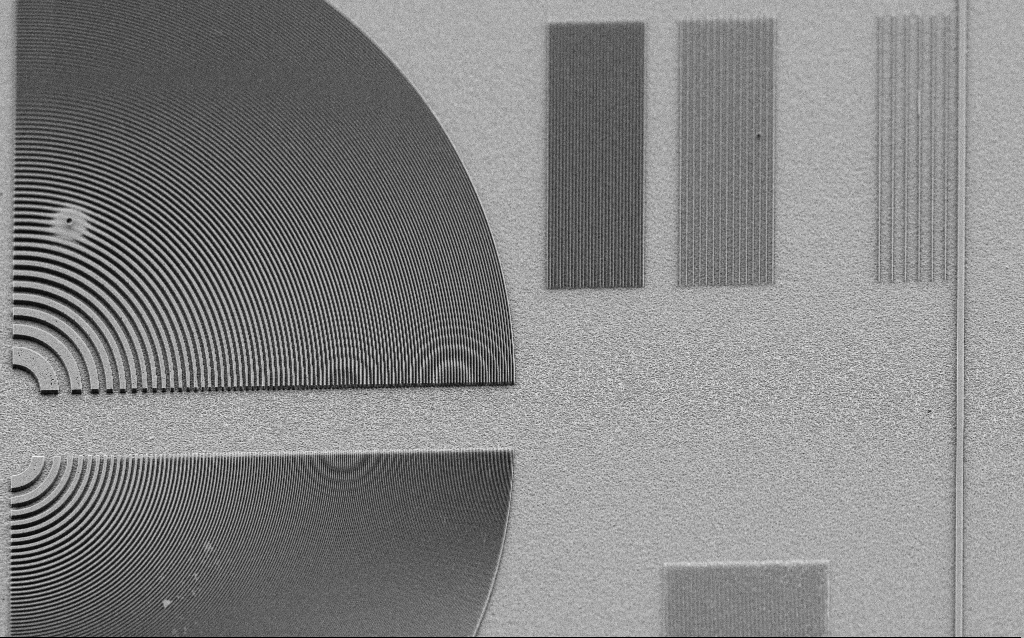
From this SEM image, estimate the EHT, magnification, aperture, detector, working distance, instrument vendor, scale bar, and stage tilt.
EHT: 3 kV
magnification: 2.48 K X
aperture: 30 µm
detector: SE2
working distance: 5 mm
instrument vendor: Zeiss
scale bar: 10000 nm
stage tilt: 45°